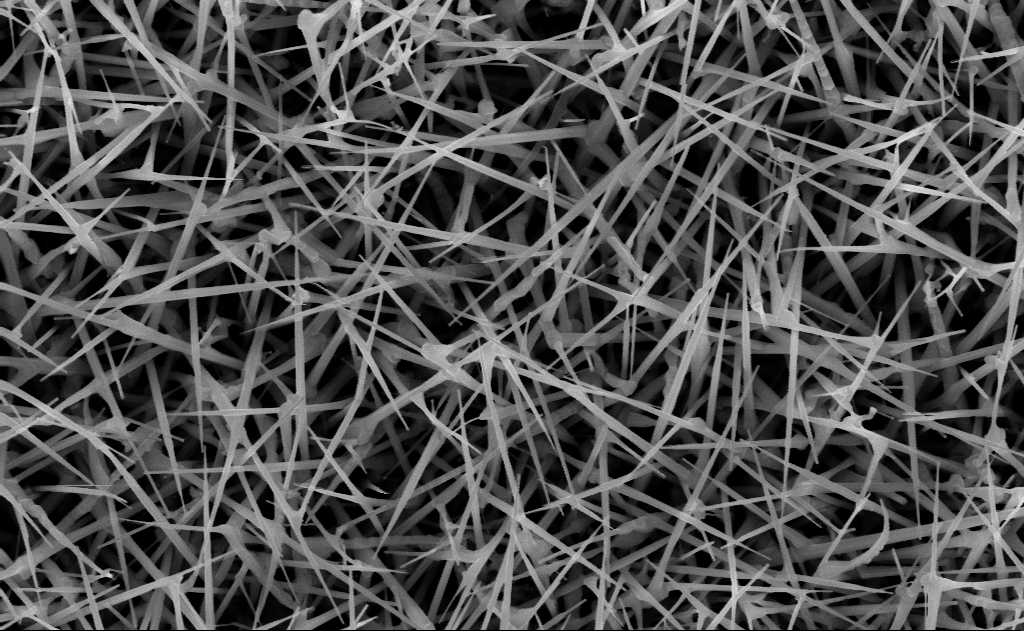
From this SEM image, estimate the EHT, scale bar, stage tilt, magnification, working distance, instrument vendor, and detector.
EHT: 10 kV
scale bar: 1000 nm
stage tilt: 0°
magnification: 40 K X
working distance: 15 mm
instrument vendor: Zeiss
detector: InLens